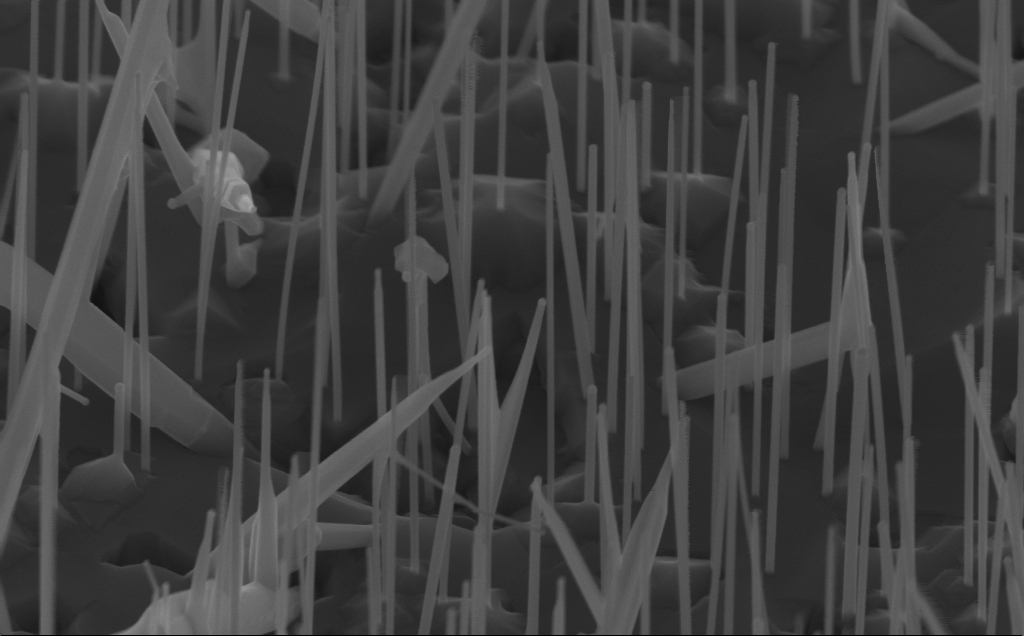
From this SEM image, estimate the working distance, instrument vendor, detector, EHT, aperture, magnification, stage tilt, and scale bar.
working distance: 5 mm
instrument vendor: Zeiss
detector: InLens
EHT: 10 kV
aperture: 30 µm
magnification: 80 K X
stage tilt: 45°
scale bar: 200 nm